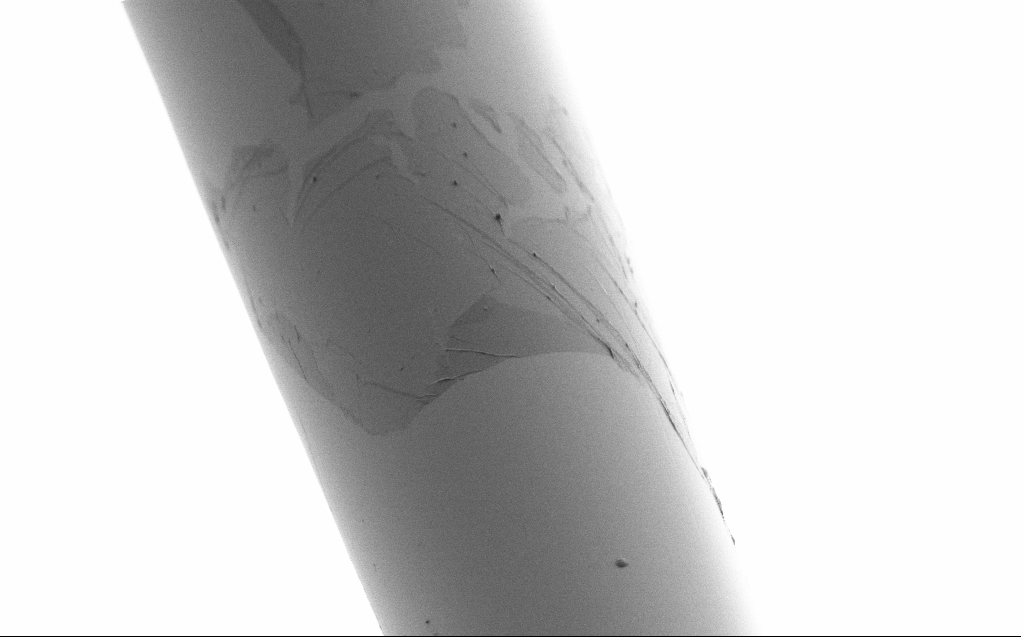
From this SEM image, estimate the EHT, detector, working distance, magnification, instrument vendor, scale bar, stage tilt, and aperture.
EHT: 1 kV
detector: SE2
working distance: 4 mm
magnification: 5 K X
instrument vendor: Zeiss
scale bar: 10000 nm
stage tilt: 45°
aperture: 30 µm